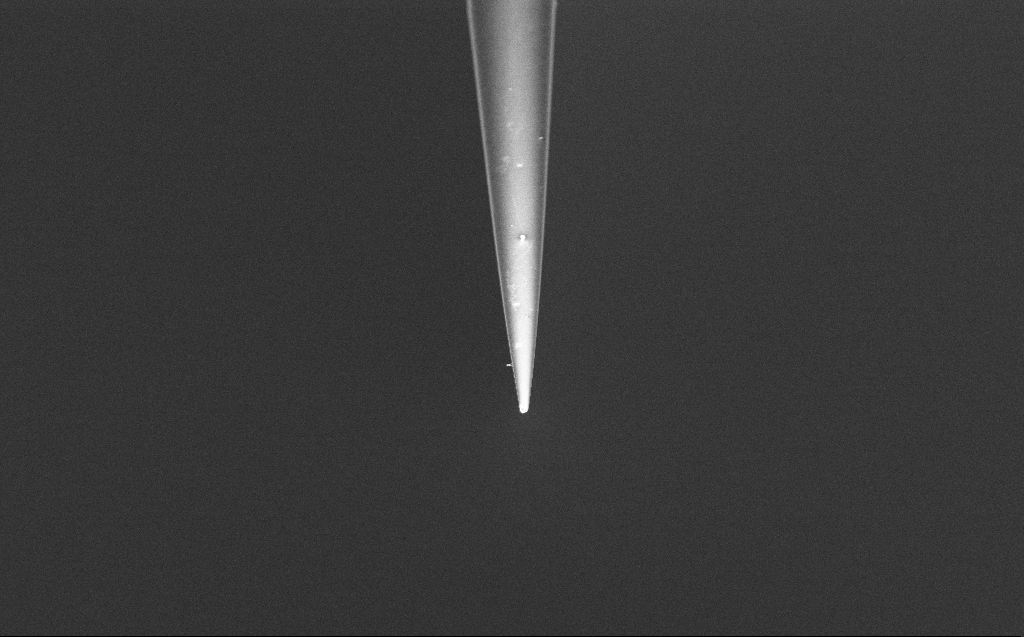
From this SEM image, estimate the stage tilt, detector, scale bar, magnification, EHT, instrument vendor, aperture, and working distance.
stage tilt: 45°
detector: InLens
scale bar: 2000 nm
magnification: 10 K X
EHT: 2 kV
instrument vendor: Zeiss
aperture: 30 µm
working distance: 6 mm